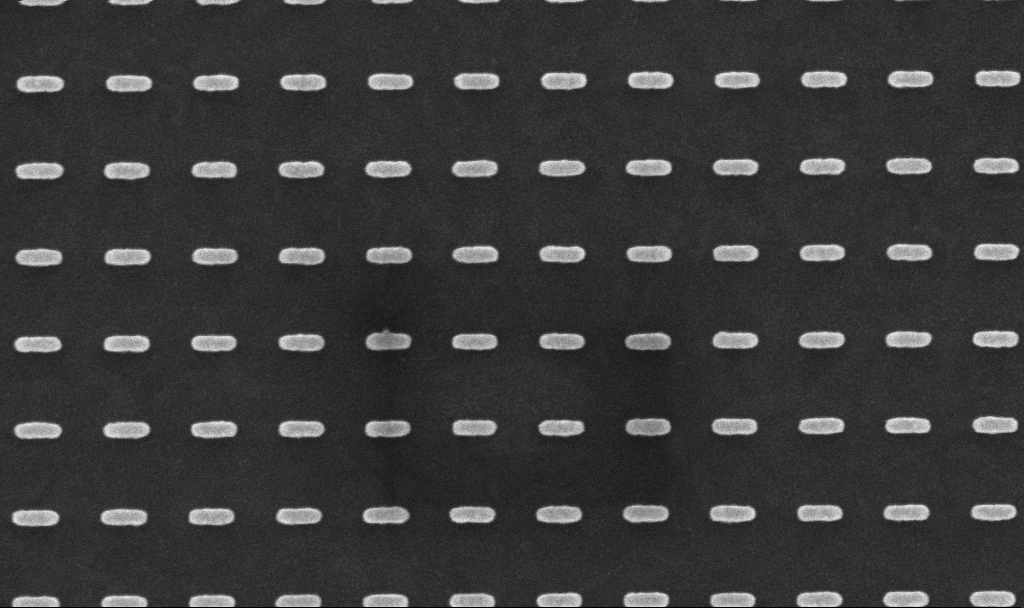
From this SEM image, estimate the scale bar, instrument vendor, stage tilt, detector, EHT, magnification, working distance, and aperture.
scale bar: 200 nm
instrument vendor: Zeiss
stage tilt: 0°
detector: InLens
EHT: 5 kV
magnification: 139.02 K X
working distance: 3.6 mm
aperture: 30 µm